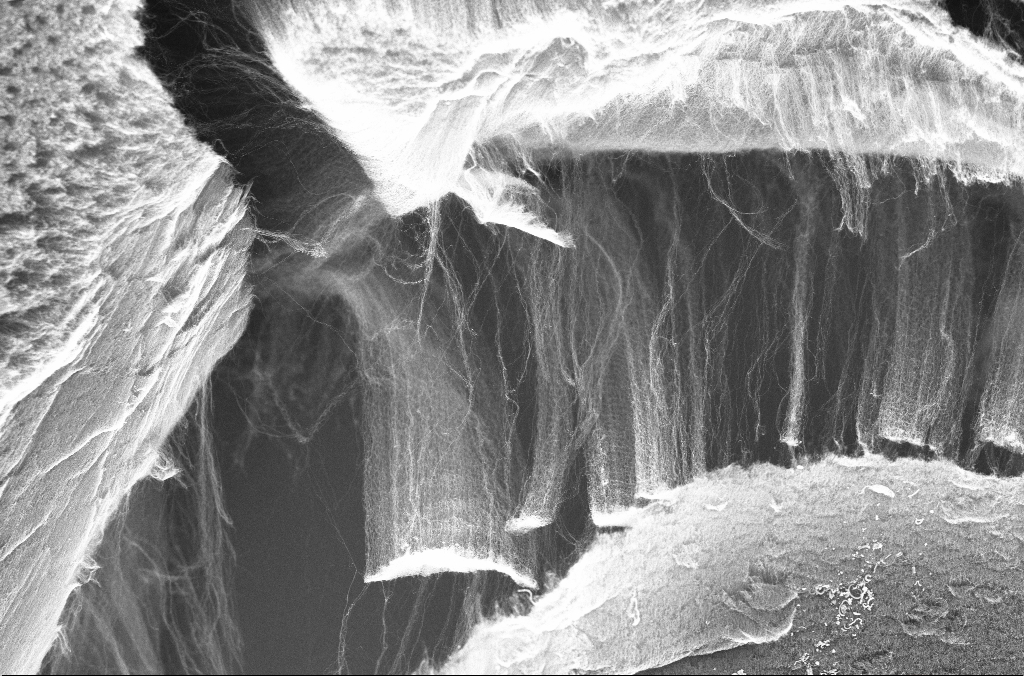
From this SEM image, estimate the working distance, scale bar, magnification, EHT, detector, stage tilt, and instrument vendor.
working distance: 3.9 mm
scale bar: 20000 nm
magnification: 3 K X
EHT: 5 kV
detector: InLens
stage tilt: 0°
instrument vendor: Zeiss